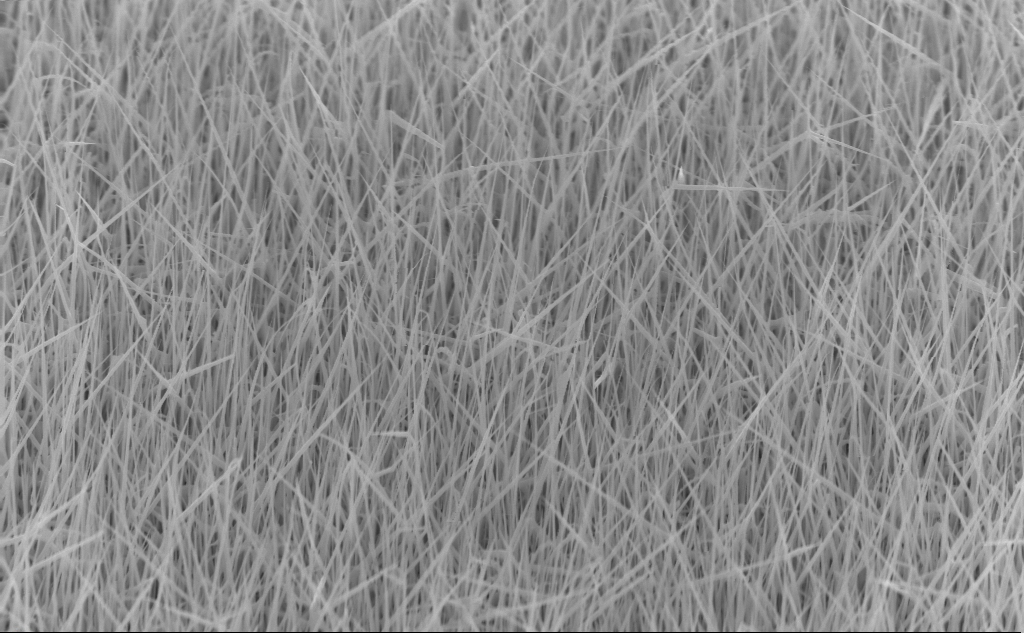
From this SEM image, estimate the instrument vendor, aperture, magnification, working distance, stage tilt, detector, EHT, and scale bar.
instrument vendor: Zeiss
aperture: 30 µm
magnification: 20 K X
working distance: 4 mm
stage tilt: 45°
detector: InLens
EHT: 10 kV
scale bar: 2000 nm